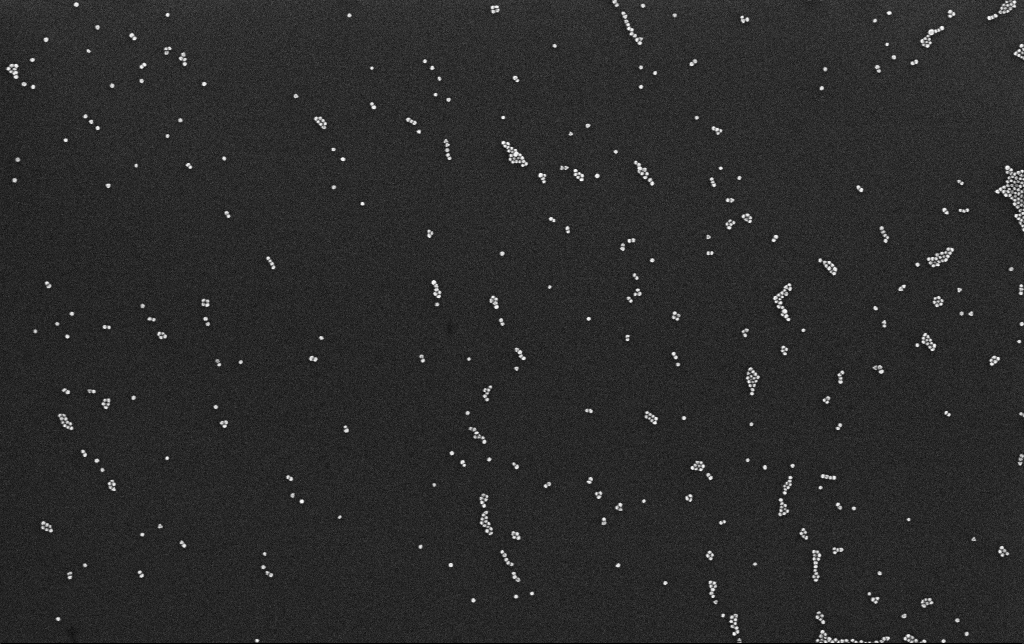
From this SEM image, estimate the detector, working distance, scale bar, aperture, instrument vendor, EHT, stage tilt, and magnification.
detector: InLens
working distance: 3.1 mm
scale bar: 200 nm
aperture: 30 µm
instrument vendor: Zeiss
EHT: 10 kV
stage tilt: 0°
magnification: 100 K X